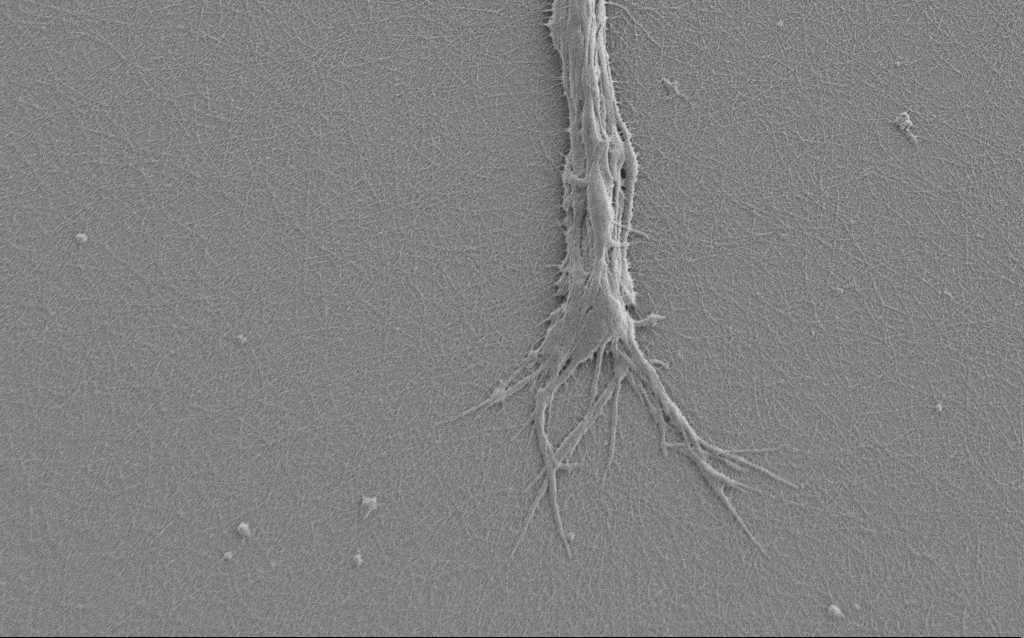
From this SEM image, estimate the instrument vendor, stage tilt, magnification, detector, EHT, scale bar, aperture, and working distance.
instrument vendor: Zeiss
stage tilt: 0°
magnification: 7.5 K X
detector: SE2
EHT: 1 kV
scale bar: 2000 nm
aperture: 30 µm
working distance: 6 mm